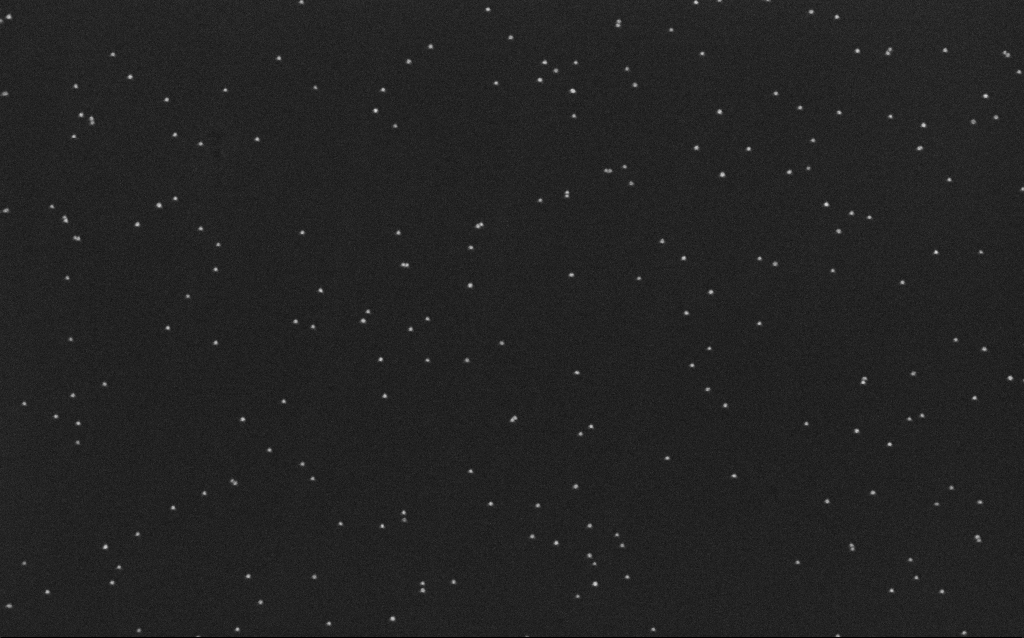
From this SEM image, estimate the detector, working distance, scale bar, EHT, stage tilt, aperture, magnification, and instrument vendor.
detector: InLens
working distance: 6.5 mm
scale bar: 200 nm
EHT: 10 kV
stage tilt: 0°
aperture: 30 µm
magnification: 100 K X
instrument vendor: Zeiss